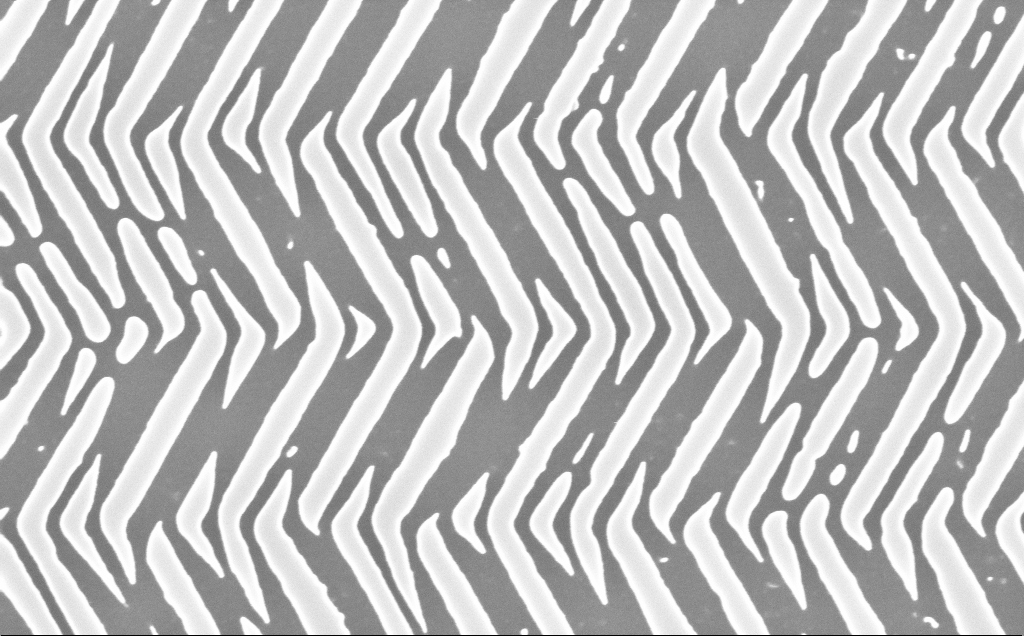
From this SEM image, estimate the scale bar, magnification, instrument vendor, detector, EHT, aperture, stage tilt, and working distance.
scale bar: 1000 nm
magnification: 71.67 K X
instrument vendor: Zeiss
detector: InLens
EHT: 10 kV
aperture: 30 µm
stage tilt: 0°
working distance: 6 mm